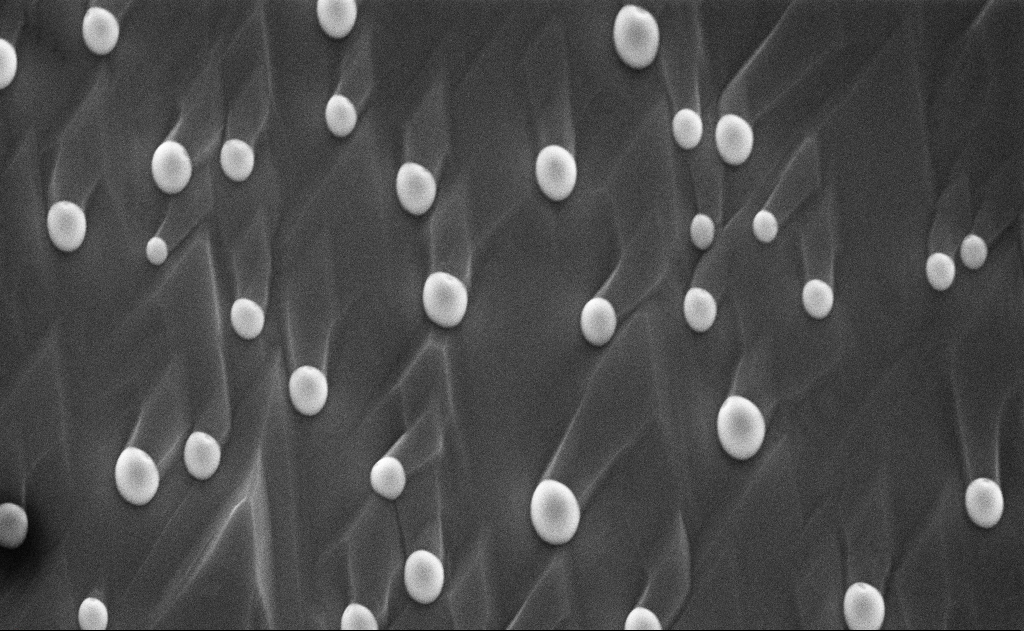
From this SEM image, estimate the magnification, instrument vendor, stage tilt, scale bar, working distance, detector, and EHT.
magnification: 20 K X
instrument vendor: Zeiss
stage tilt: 0°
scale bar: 2000 nm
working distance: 12 mm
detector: InLens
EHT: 10 kV